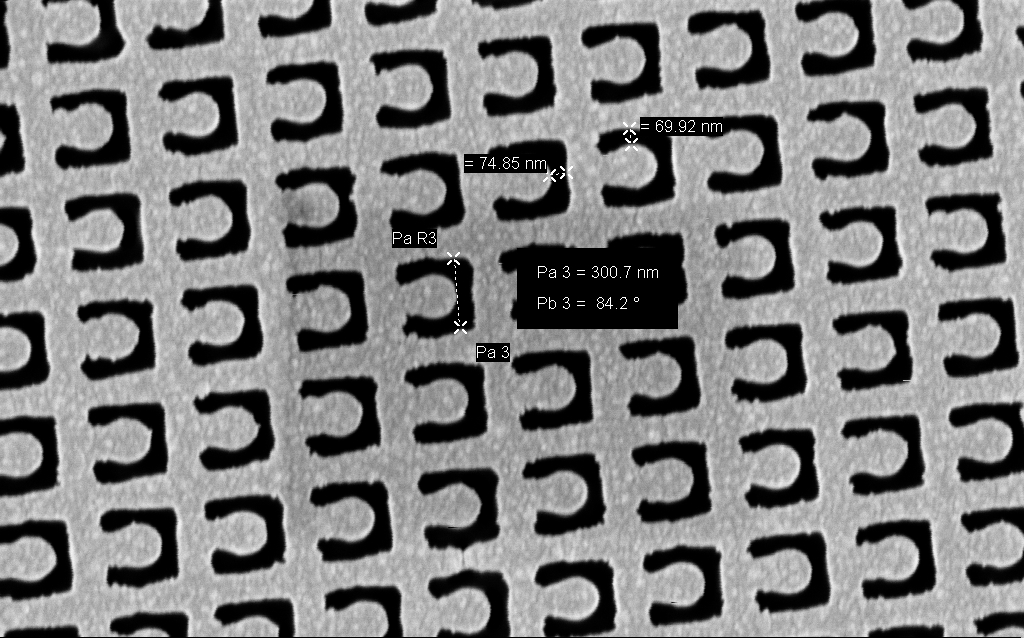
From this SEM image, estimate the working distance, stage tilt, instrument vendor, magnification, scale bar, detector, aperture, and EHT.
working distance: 6.5 mm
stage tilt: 0°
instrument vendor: Zeiss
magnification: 84.68 K X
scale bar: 200 nm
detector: InLens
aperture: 30 µm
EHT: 1.5 kV